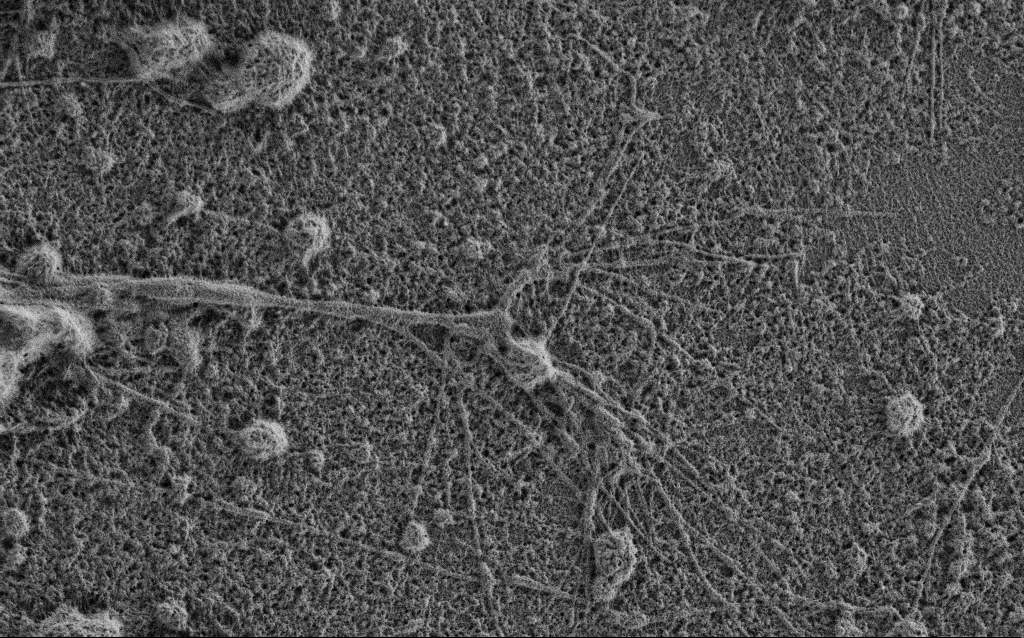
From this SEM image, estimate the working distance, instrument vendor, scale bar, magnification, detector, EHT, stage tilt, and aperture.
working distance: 3.4 mm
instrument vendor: Zeiss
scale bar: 2000 nm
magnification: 10 K X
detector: SE2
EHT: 0.9 kV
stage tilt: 0°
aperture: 30 µm